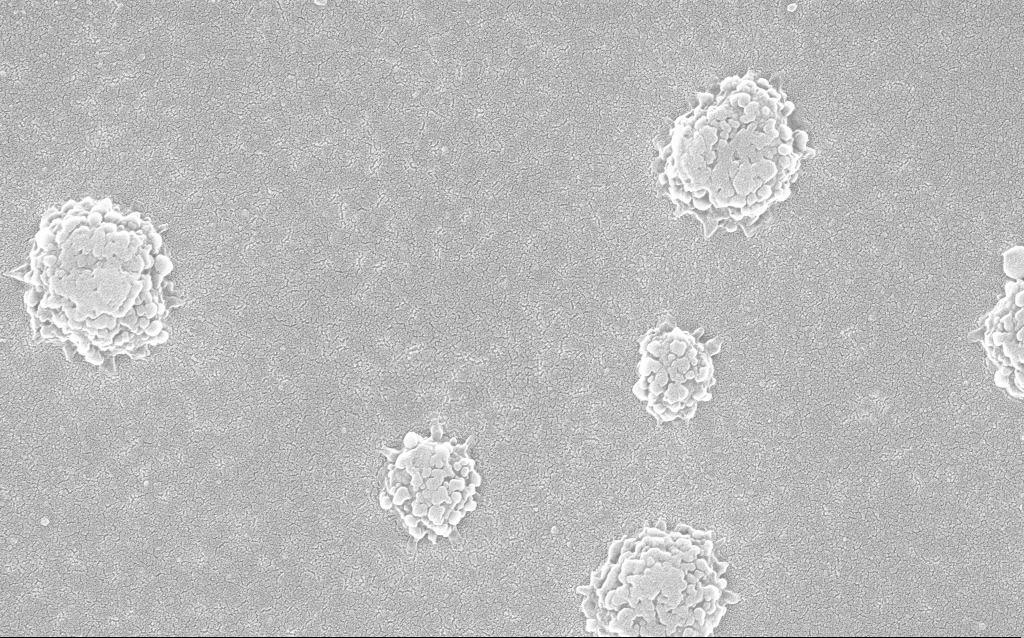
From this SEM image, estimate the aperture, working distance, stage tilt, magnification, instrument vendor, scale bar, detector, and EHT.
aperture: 30 µm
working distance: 1.8 mm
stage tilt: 0°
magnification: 100 K X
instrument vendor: Zeiss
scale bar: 200 nm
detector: InLens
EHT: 20 kV